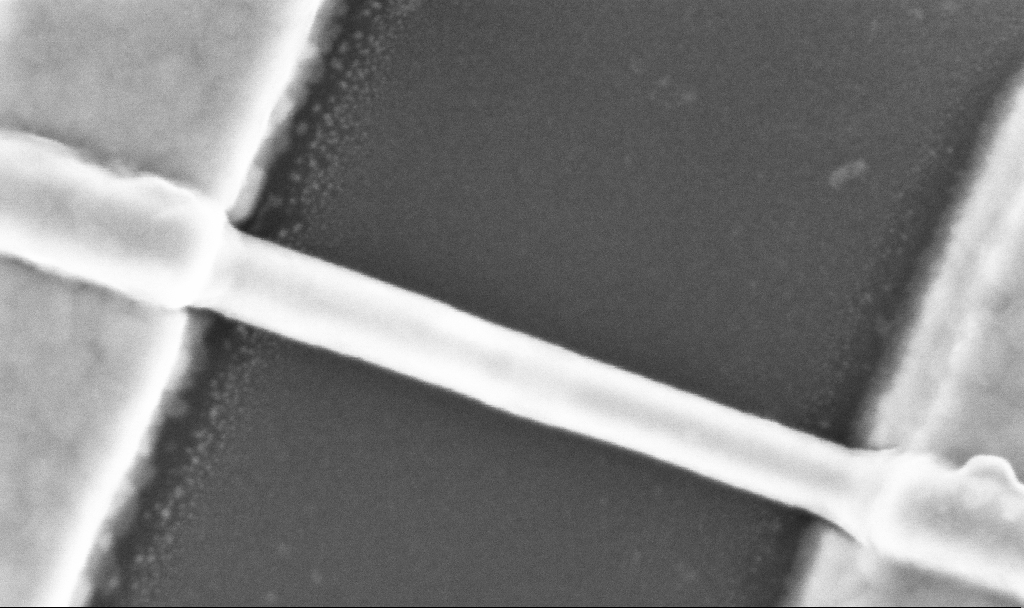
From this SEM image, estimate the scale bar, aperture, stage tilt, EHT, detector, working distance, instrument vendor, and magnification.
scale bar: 100 nm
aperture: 30 µm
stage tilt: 0°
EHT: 5 kV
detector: InLens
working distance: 6.7 mm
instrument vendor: Zeiss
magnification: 300 K X